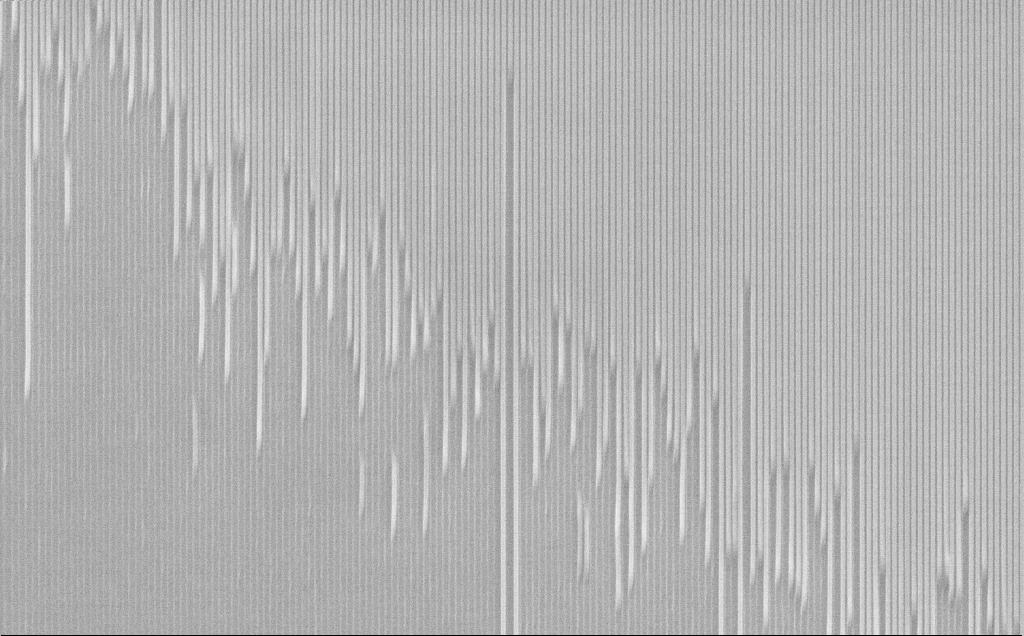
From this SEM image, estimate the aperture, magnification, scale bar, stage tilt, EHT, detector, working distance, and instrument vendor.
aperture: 30 µm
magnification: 13.17 K X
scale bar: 2000 nm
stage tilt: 45°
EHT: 10 kV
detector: SE2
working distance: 8 mm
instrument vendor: Zeiss